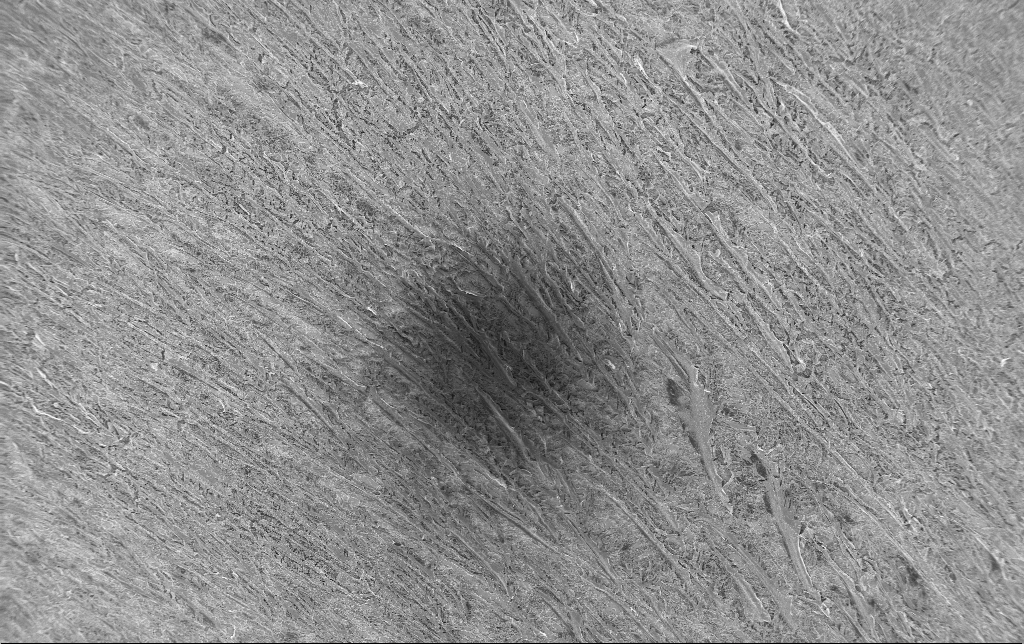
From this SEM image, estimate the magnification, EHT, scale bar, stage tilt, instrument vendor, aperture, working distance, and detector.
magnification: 0.2 K X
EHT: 1.5 kV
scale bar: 100000 nm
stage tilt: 0°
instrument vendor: Zeiss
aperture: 30 µm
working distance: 3.2 mm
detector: InLens